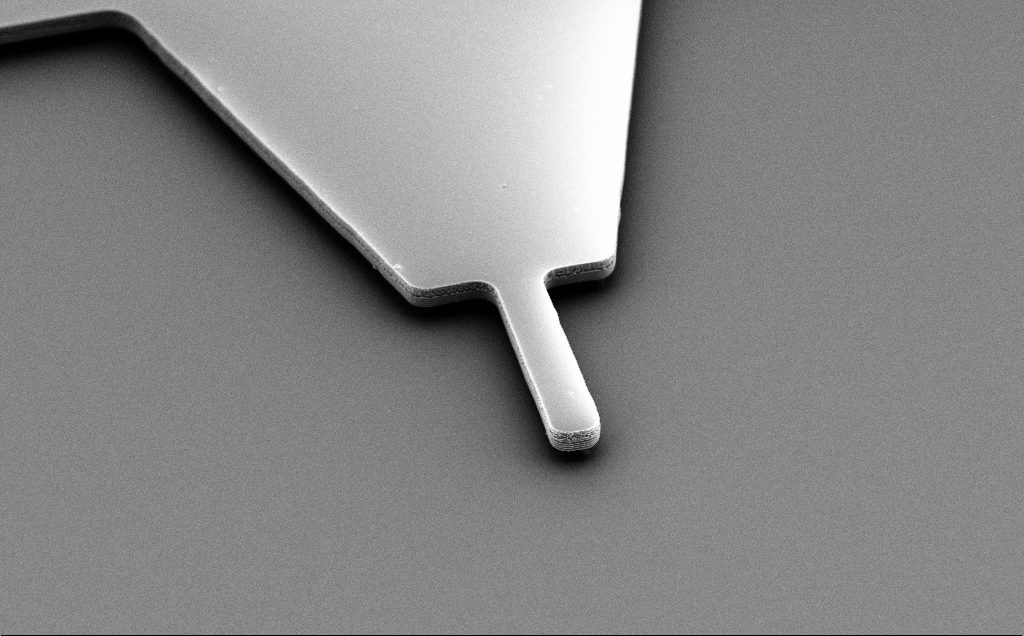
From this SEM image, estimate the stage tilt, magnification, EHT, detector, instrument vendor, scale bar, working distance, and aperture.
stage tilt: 50°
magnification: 3.78 K X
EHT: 5 kV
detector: SE2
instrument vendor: Zeiss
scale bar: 10000 nm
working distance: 10 mm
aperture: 30 µm